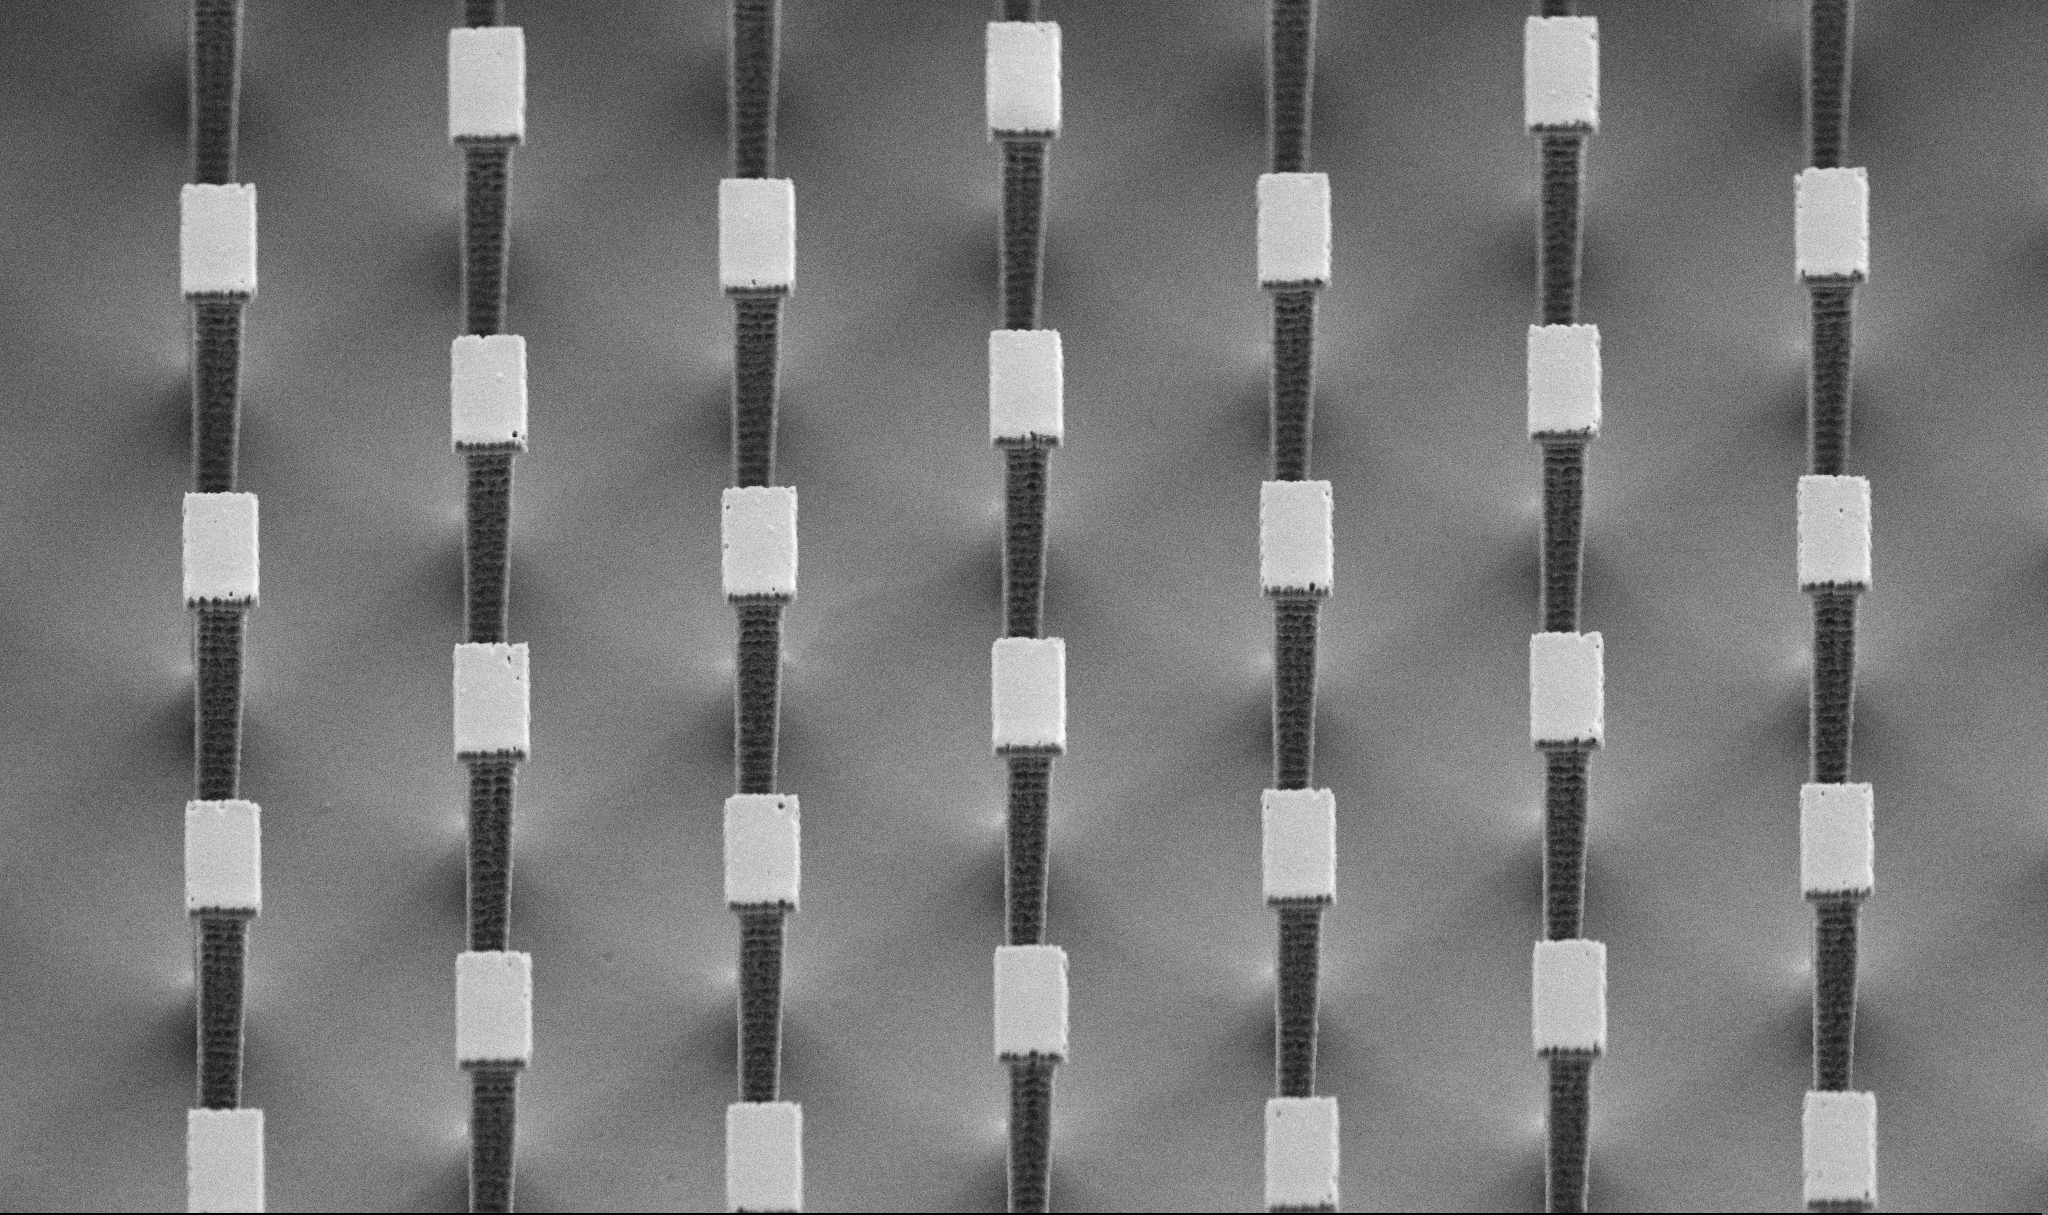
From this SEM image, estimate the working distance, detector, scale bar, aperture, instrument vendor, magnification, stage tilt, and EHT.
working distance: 6.5 mm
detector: SE2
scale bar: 10000 nm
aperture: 30 µm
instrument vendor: Zeiss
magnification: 4.73 K X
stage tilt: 45°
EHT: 5 kV